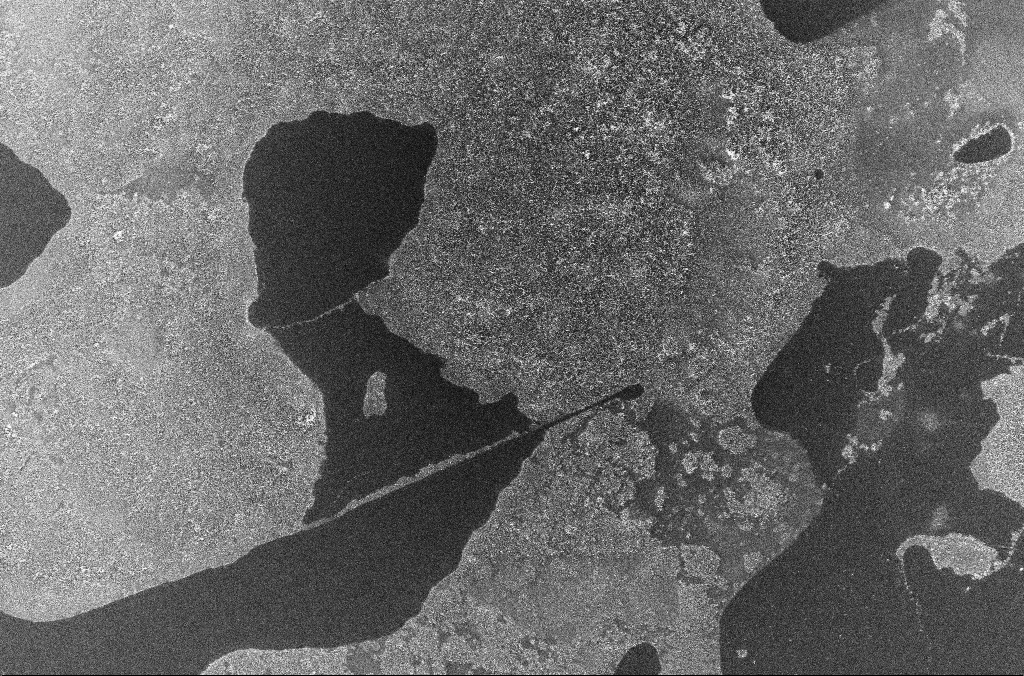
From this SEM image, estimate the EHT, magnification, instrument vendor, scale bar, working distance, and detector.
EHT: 10 kV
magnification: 0.5 K X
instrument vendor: Zeiss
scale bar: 100000 nm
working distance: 3.3 mm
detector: InLens